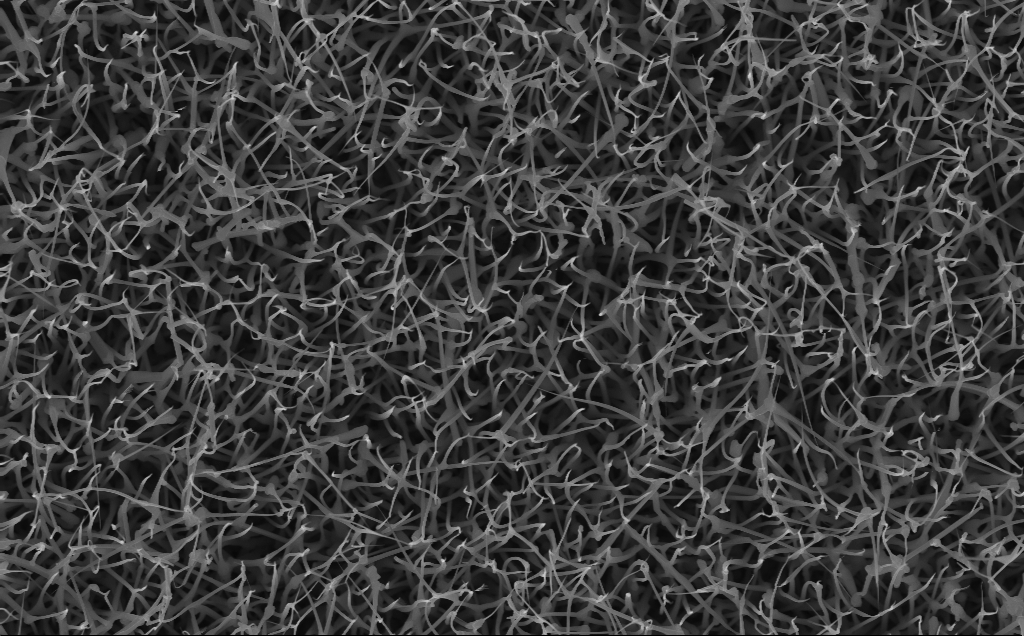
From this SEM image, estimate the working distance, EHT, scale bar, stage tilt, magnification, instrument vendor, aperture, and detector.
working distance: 4 mm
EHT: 10 kV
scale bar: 2000 nm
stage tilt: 0°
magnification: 20 K X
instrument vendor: Zeiss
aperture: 30 µm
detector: InLens